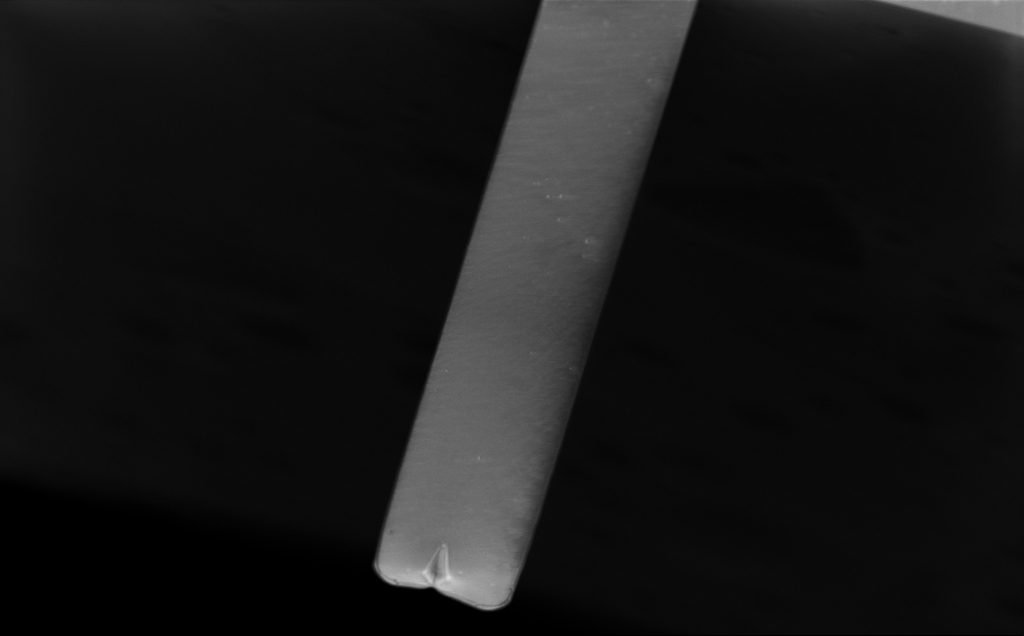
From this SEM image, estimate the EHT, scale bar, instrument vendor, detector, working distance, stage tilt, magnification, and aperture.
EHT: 5 kV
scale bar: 20000 nm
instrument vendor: Zeiss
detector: InLens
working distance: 7 mm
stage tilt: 0°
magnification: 1.49 K X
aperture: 30 µm